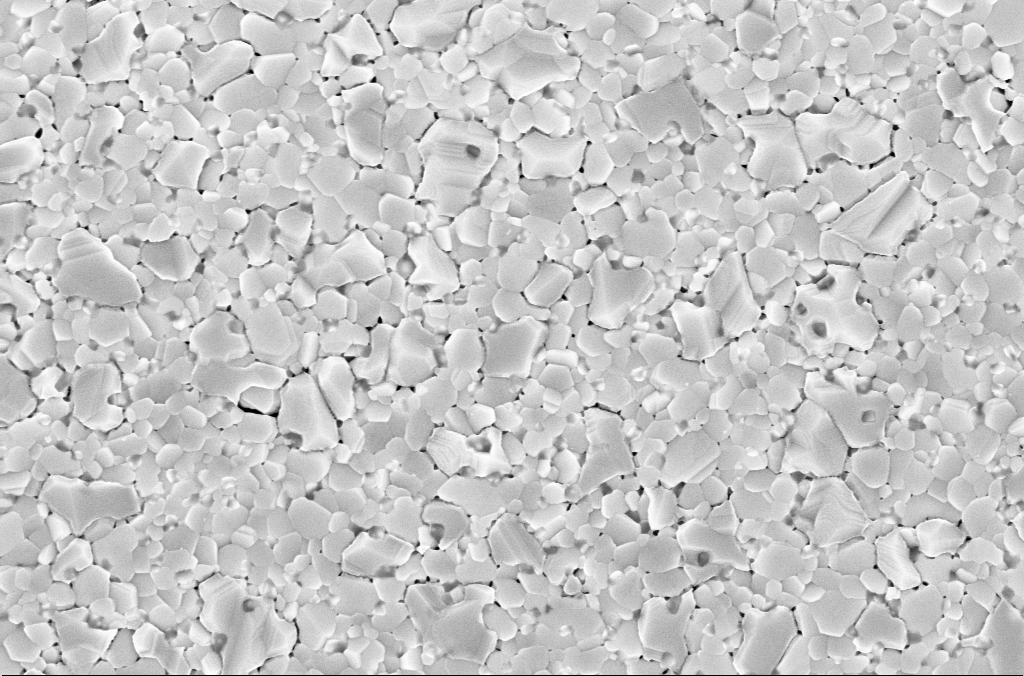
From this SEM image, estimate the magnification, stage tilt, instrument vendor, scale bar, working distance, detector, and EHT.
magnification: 50 K X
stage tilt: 0°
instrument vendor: Zeiss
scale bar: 1000 nm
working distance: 3 mm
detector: InLens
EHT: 5 kV